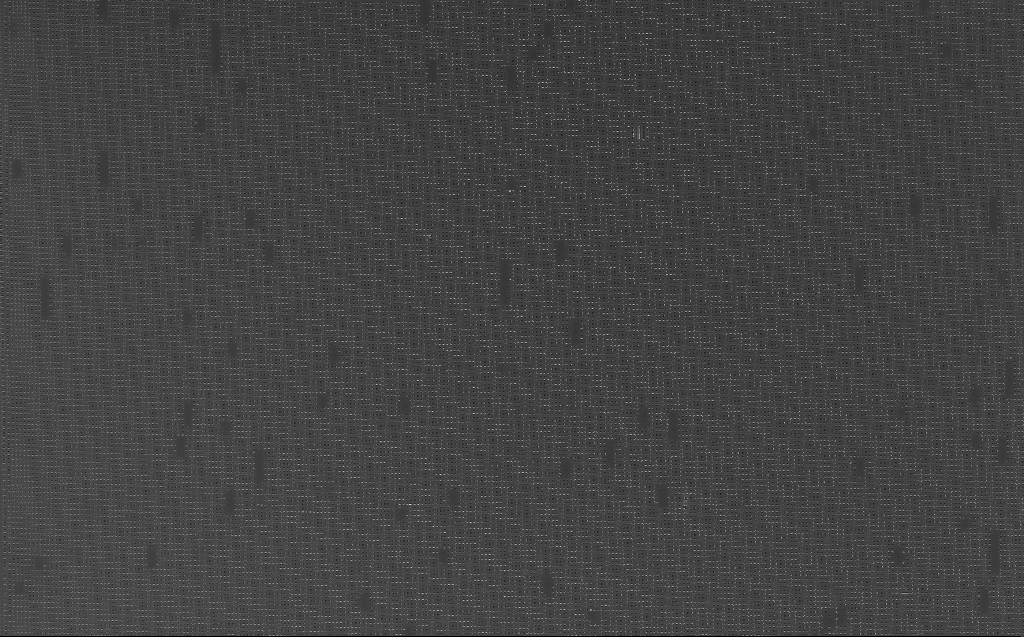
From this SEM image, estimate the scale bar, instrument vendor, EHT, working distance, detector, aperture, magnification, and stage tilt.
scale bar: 10000 nm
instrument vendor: Zeiss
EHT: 10 kV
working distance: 6 mm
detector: InLens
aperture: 30 µm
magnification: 2.78 K X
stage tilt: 0°